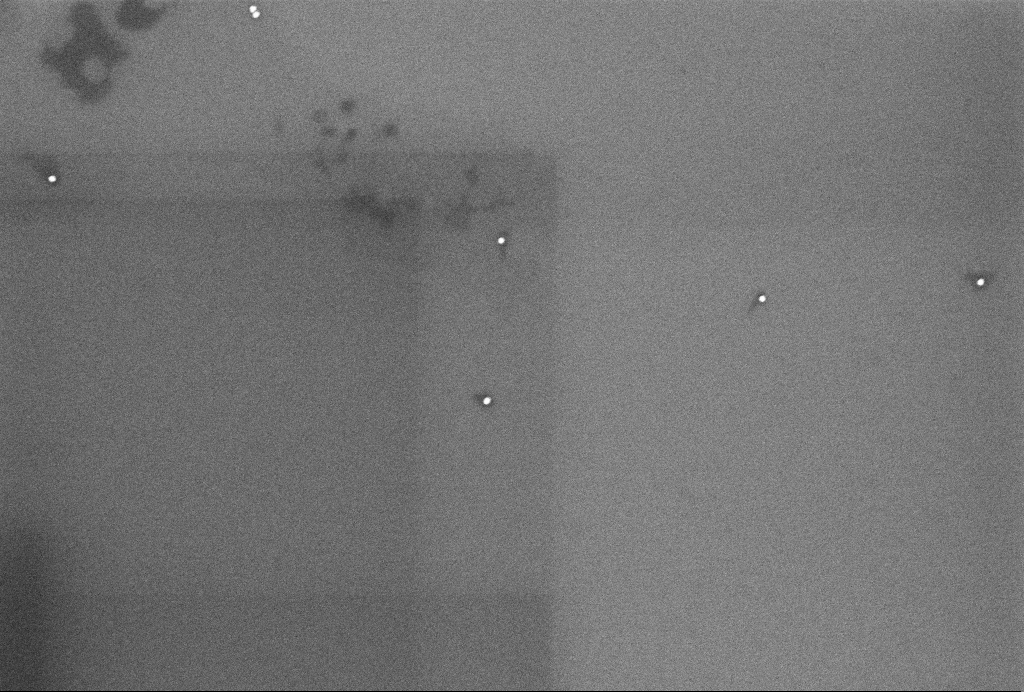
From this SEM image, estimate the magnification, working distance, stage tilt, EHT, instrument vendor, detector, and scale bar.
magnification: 95 K X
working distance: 3.2 mm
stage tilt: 0°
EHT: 4 kV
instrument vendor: Zeiss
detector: InLens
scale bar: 200 nm